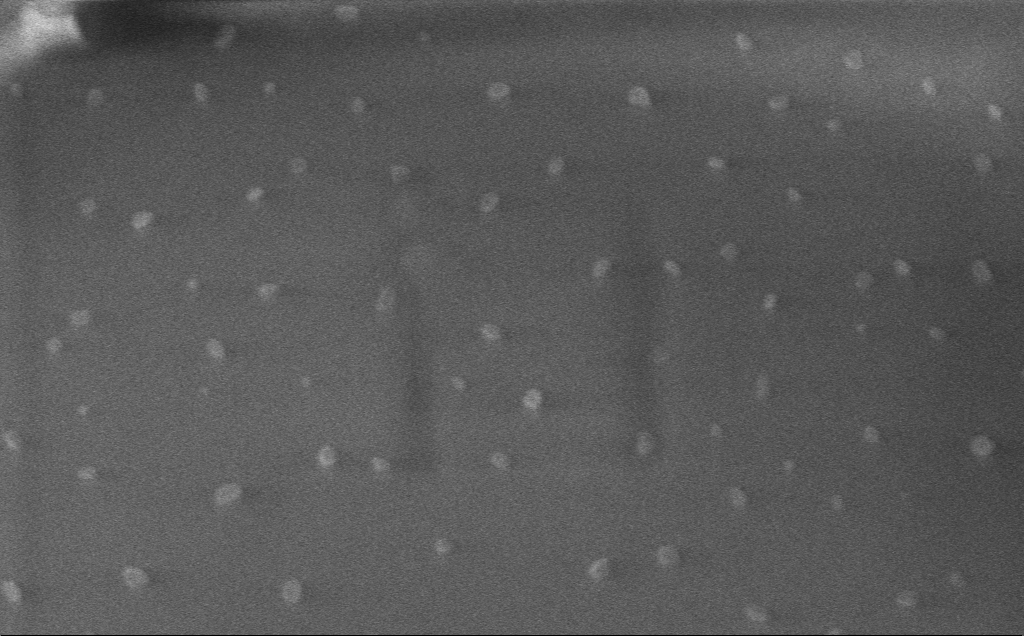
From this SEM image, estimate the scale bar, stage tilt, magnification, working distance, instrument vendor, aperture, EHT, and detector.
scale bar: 200 nm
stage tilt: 0°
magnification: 96.74 K X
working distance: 3 mm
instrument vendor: Zeiss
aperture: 30 µm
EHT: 1 kV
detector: InLens